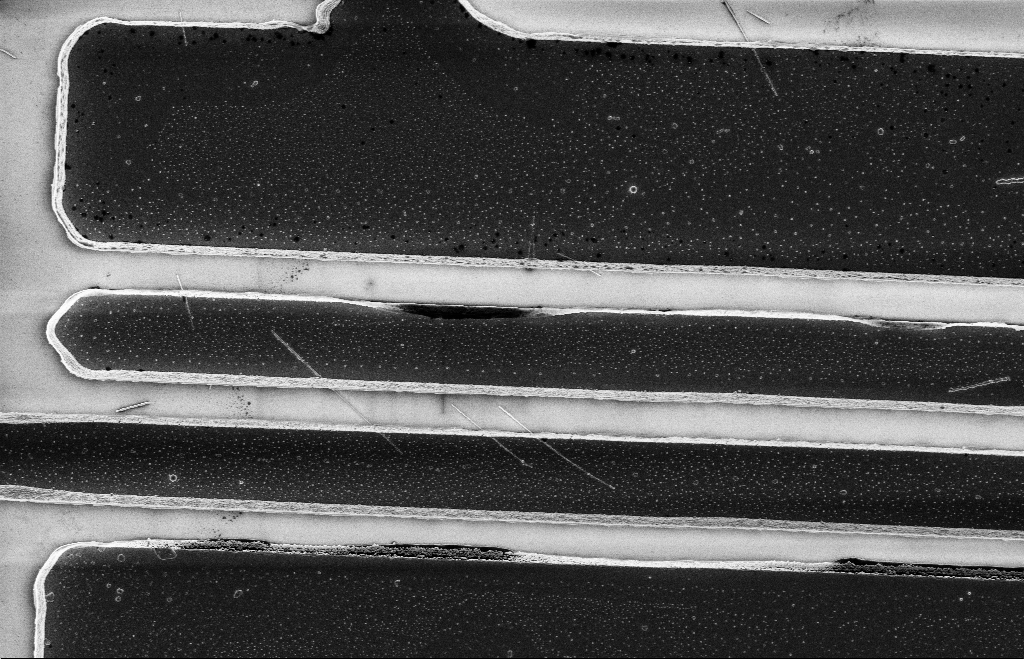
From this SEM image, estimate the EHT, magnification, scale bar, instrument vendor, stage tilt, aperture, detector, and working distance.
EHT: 5 kV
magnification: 7.71 K X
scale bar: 2000 nm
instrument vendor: Zeiss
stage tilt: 0°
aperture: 20 µm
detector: InLens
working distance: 12 mm